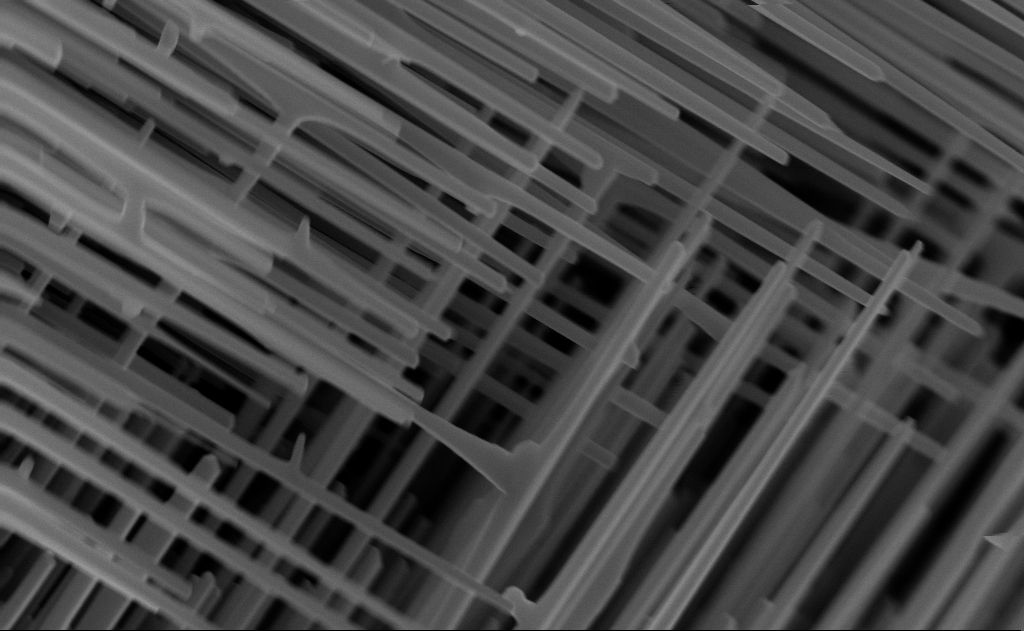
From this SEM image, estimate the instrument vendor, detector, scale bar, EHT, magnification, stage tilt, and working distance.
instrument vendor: Zeiss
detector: InLens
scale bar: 1000 nm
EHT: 10 kV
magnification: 40 K X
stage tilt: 0°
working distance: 6 mm